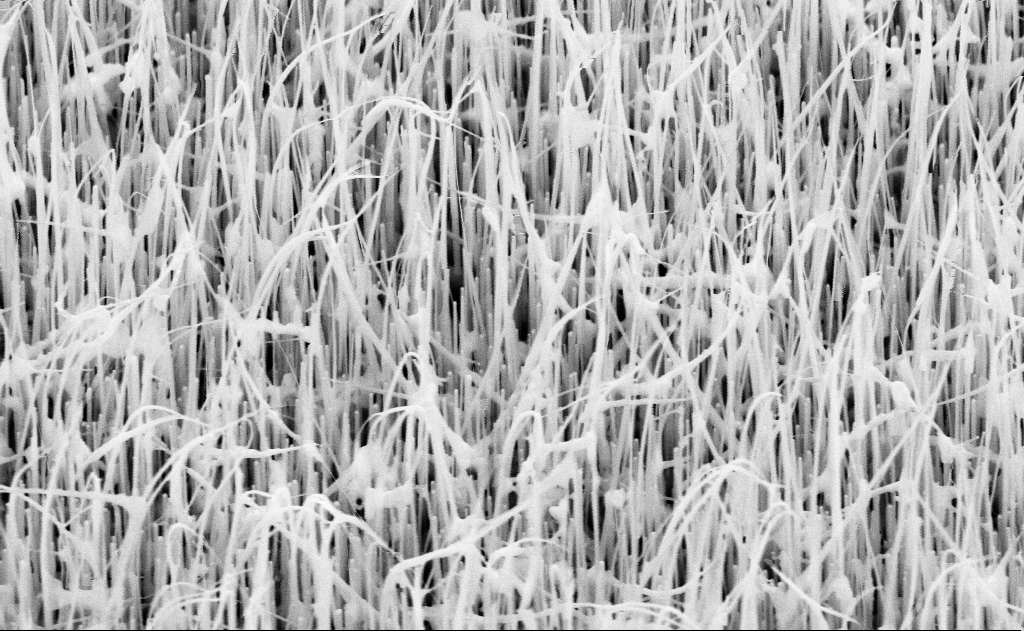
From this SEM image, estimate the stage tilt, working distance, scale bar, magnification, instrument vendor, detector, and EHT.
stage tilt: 45°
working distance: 14 mm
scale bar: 1000 nm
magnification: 40 K X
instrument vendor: Zeiss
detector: SE2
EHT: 10 kV